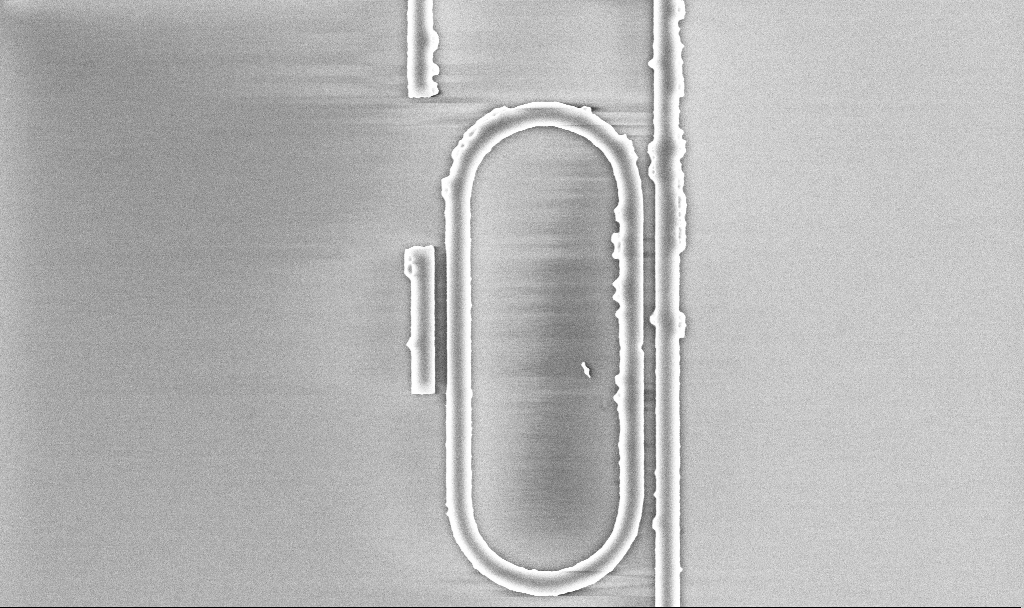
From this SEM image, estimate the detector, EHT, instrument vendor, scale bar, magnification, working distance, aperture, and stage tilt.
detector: InLens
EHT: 5 kV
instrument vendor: Zeiss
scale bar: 2000 nm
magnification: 18.43 K X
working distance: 5.2 mm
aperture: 30 µm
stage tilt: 0°